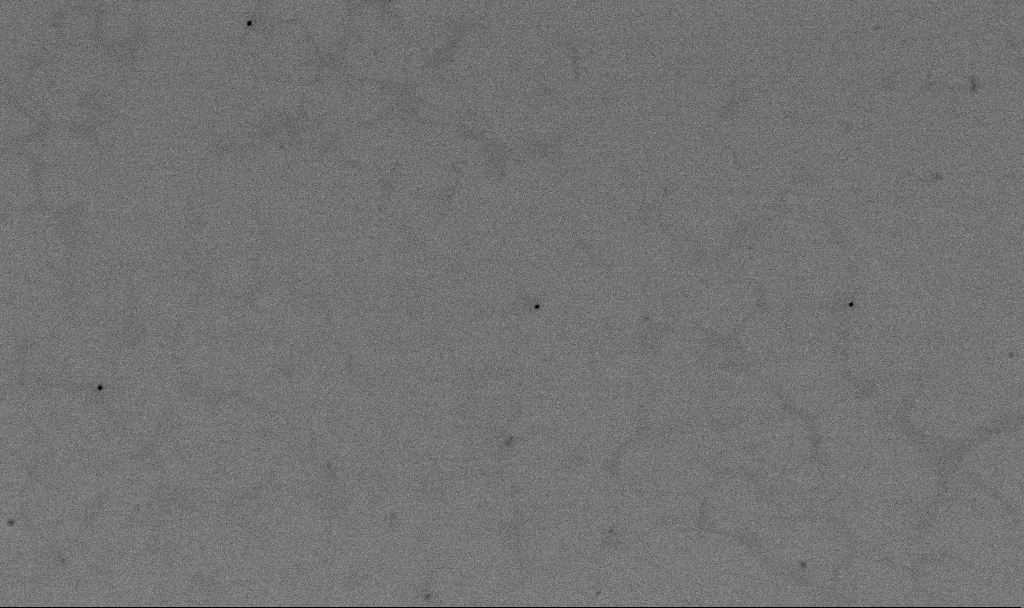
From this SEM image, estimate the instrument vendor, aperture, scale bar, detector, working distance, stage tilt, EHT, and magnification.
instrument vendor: Zeiss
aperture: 30 µm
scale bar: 1000 nm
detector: SE2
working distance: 5.5 mm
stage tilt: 0°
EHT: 2 kV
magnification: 60 K X